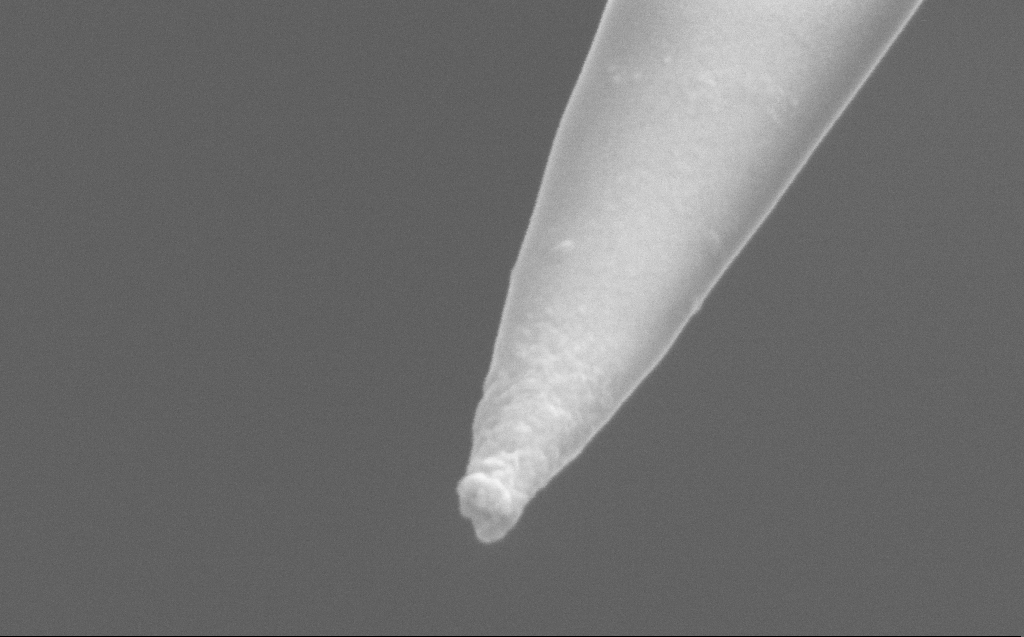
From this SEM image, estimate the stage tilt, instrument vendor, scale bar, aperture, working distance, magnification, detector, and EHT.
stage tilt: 45°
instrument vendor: Zeiss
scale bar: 200 nm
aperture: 30 µm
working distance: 6 mm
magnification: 250 K X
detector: InLens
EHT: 5 kV